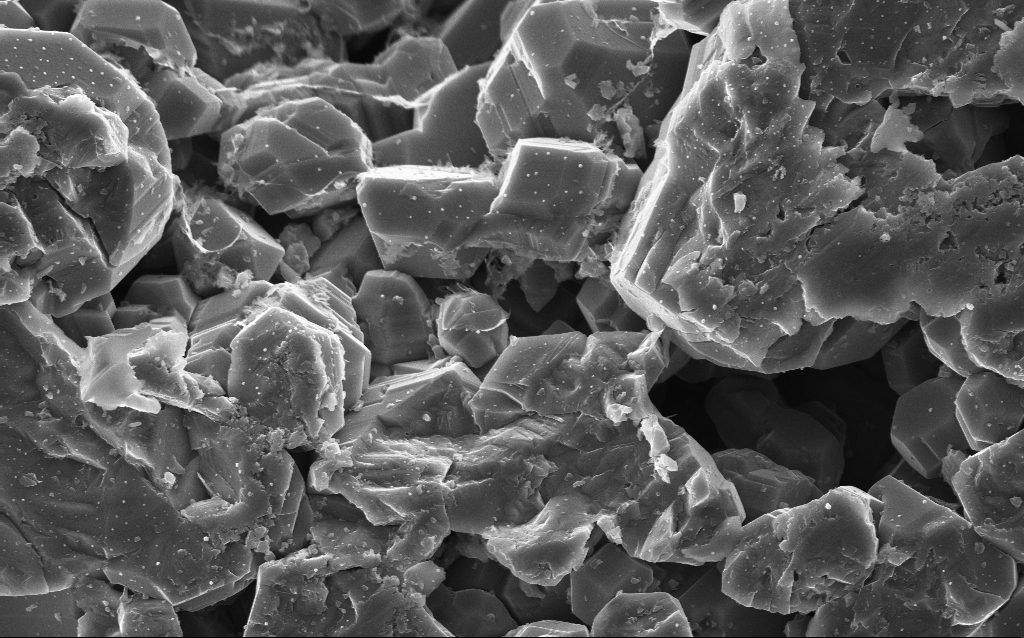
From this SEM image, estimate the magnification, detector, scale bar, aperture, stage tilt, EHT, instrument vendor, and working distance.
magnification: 10 K X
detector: InLens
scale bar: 2000 nm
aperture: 30 µm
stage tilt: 0°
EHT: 10 kV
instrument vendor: Zeiss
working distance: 3 mm